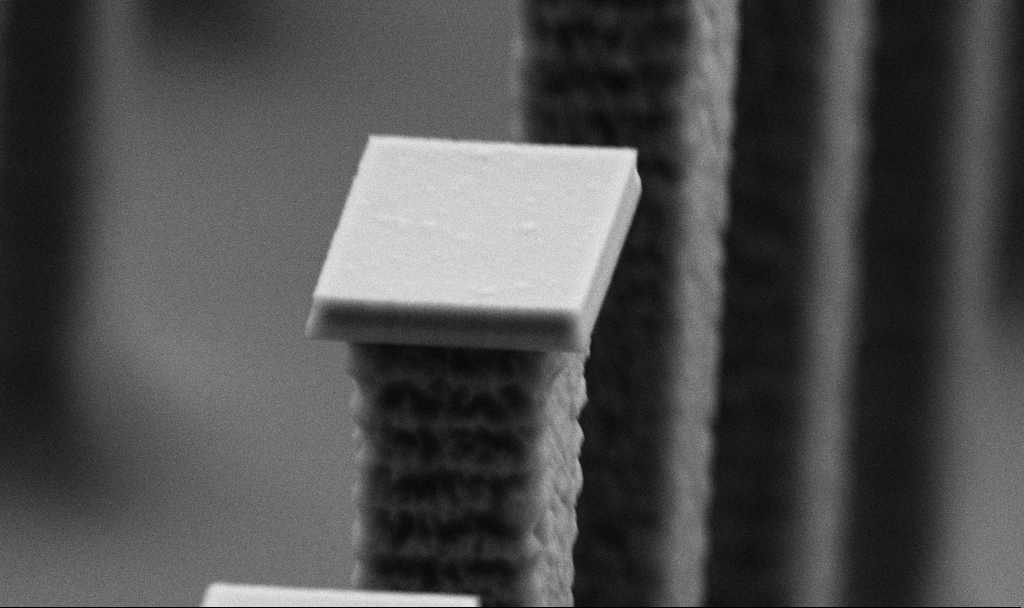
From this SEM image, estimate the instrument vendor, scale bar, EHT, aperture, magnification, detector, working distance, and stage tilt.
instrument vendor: Zeiss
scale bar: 1000 nm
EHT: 5 kV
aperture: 30 µm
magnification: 34.32 K X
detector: SE2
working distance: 5.6 mm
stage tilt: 70°